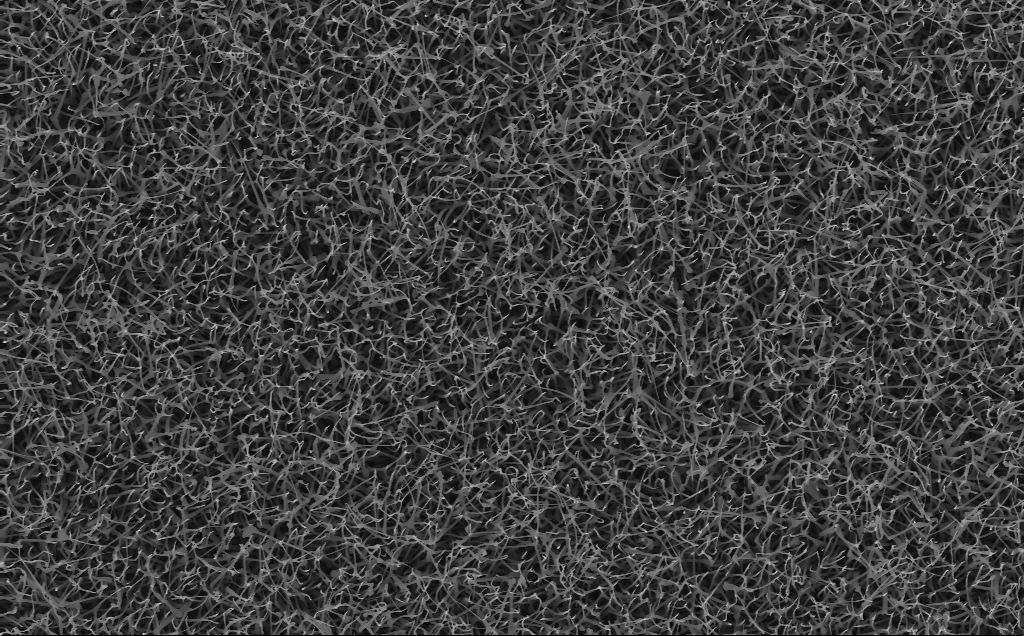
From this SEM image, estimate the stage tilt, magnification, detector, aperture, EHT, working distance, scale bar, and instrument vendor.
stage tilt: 0°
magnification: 10 K X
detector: InLens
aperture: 30 µm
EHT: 10 kV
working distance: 4 mm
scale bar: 2000 nm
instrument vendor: Zeiss